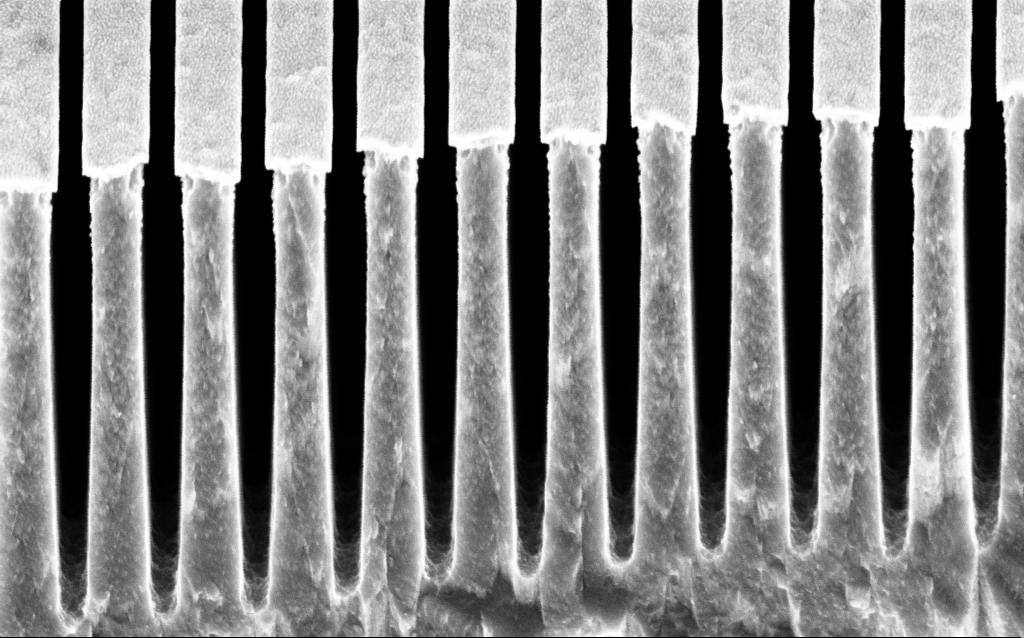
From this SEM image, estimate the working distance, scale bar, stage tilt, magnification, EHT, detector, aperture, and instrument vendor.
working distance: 6 mm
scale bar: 1000 nm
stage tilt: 45°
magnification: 67.6 K X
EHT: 3 kV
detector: InLens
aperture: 30 µm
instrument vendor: Zeiss